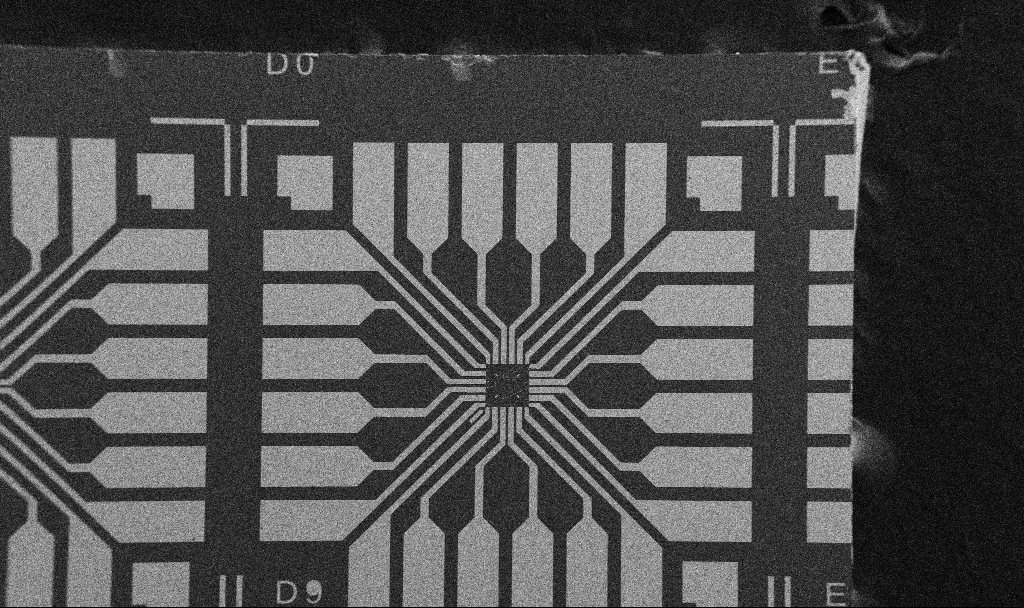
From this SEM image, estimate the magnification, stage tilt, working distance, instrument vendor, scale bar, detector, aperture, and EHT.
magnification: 0.1 K X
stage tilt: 0°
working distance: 10.7 mm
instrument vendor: Zeiss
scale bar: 200000 nm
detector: SE2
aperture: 30 µm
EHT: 5 kV